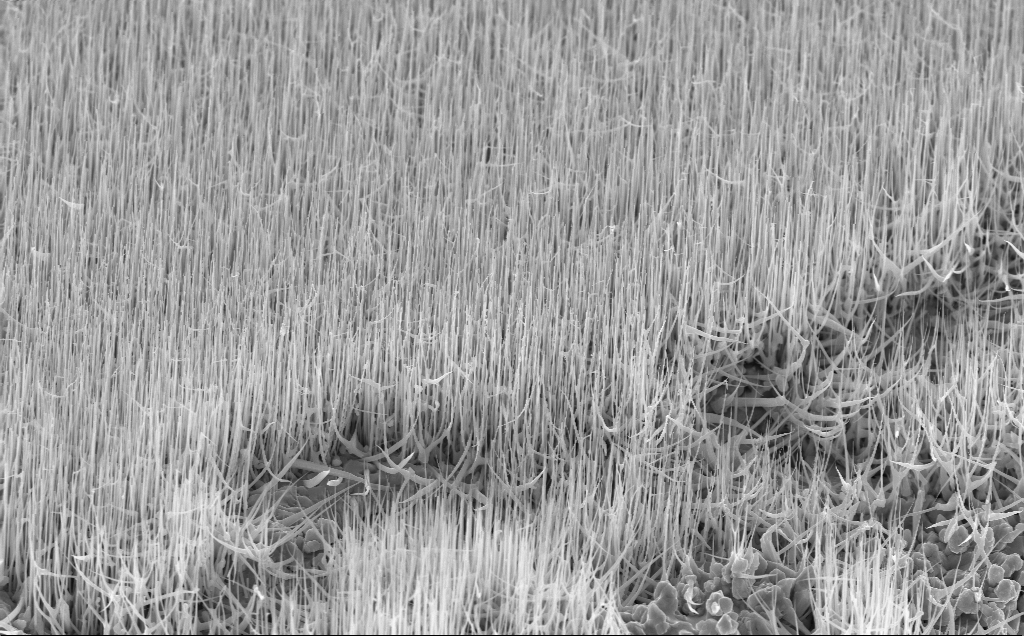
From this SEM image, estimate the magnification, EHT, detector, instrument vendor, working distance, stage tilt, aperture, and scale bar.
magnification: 10 K X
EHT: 10 kV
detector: InLens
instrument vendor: Zeiss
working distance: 6 mm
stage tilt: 45°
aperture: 30 µm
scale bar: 2000 nm